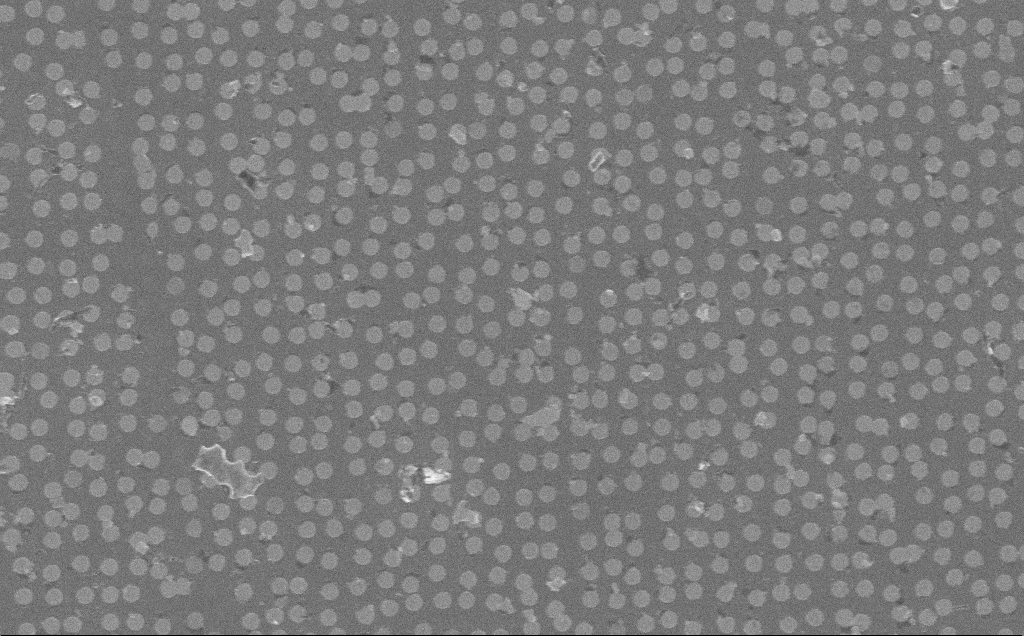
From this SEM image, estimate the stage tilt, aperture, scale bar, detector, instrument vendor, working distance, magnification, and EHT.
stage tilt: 0°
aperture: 30 µm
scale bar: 2000 nm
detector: InLens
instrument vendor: Zeiss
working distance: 7 mm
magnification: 25.72 K X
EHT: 10 kV